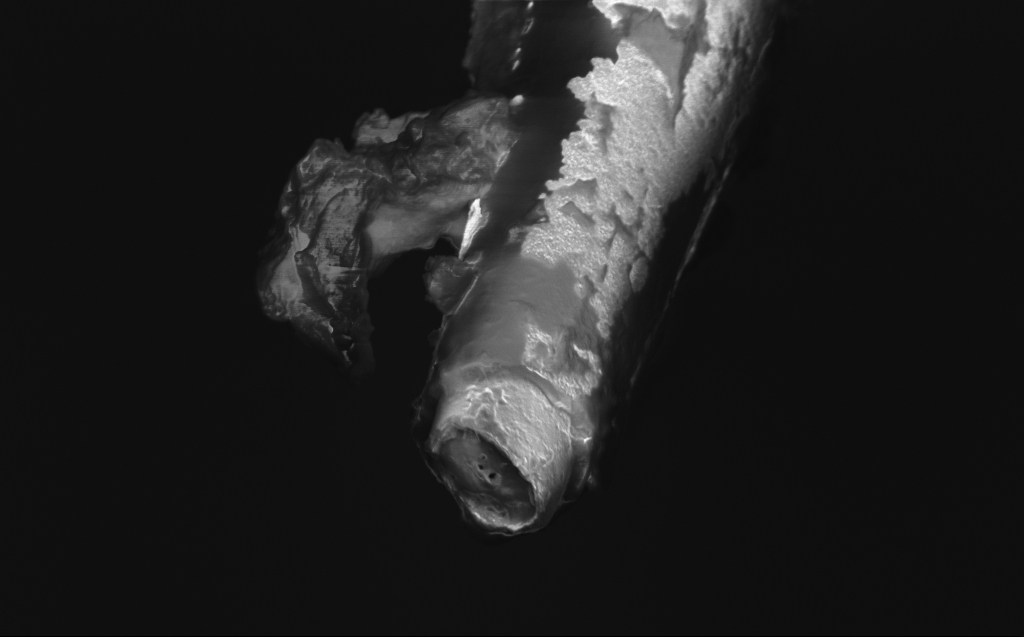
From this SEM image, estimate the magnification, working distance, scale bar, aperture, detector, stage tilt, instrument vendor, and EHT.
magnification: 20.38 K X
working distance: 4 mm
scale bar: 1000 nm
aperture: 30 µm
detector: InLens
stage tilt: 45°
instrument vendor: Zeiss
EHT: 1 kV